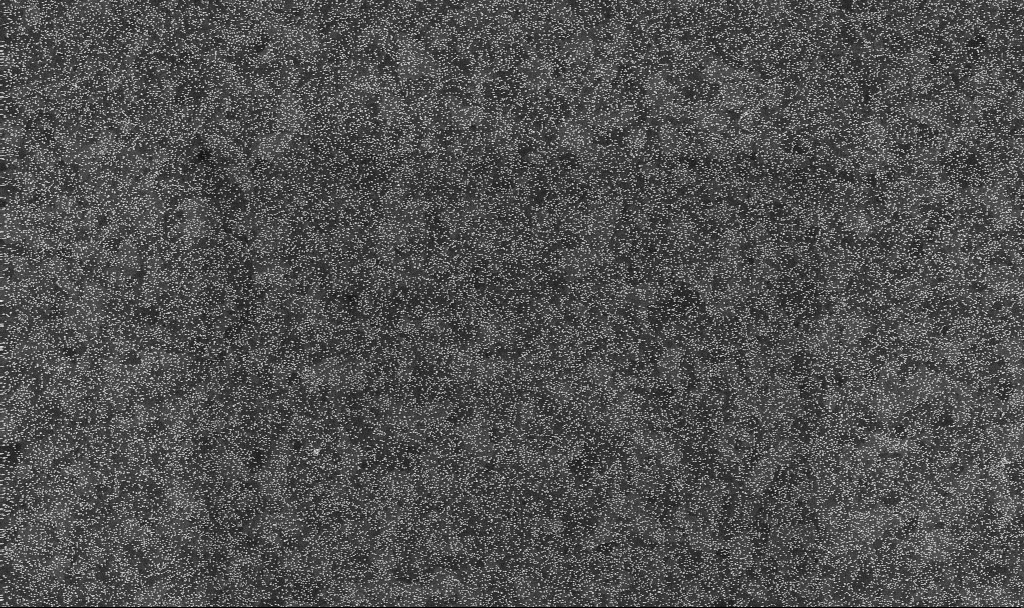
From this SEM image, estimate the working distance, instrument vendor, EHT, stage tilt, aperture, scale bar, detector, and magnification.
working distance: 3.3 mm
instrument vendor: Zeiss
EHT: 10 kV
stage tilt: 0°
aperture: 30 µm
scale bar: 1000 nm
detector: InLens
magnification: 30 K X